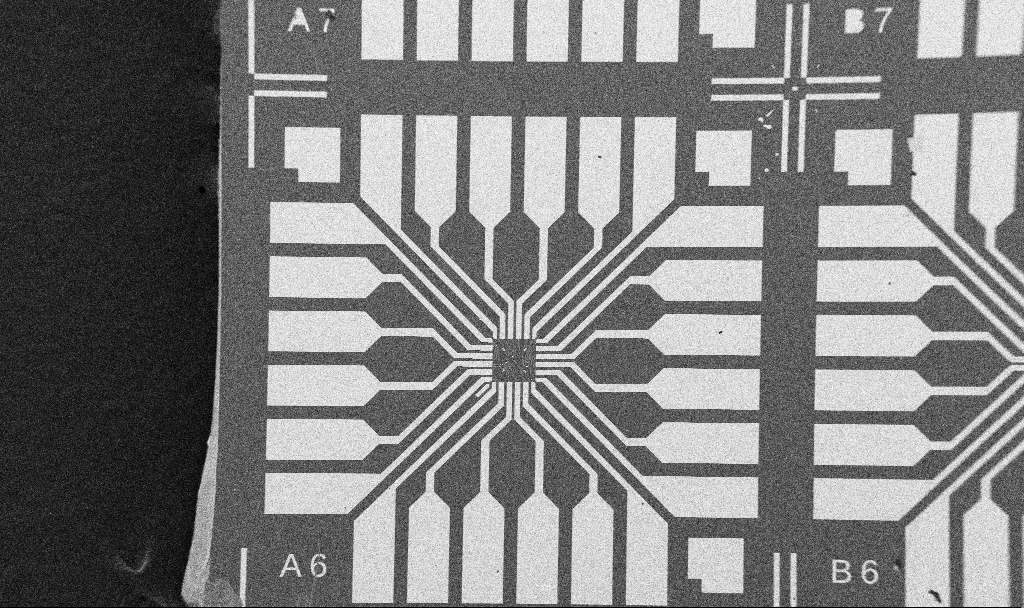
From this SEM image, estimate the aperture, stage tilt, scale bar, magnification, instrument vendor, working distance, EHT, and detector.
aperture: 30 µm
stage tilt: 0°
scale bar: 200000 nm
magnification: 0.1 K X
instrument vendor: Zeiss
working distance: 10.7 mm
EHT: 5 kV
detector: SE2